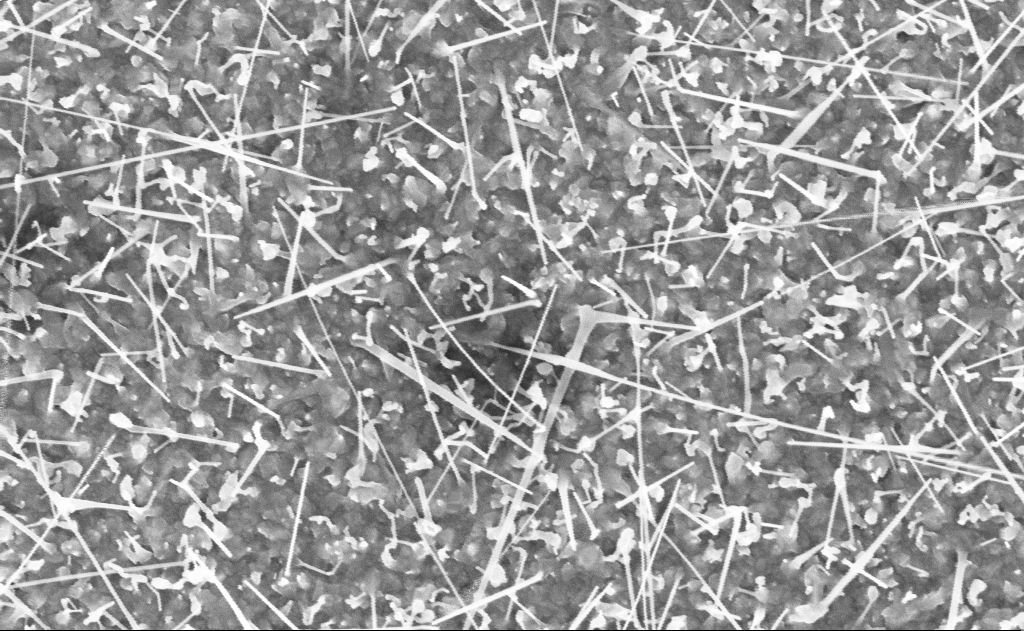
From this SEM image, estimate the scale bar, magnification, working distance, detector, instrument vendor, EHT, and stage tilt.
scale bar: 1000 nm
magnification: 40 K X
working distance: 20 mm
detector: InLens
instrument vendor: Zeiss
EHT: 10 kV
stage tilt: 0°